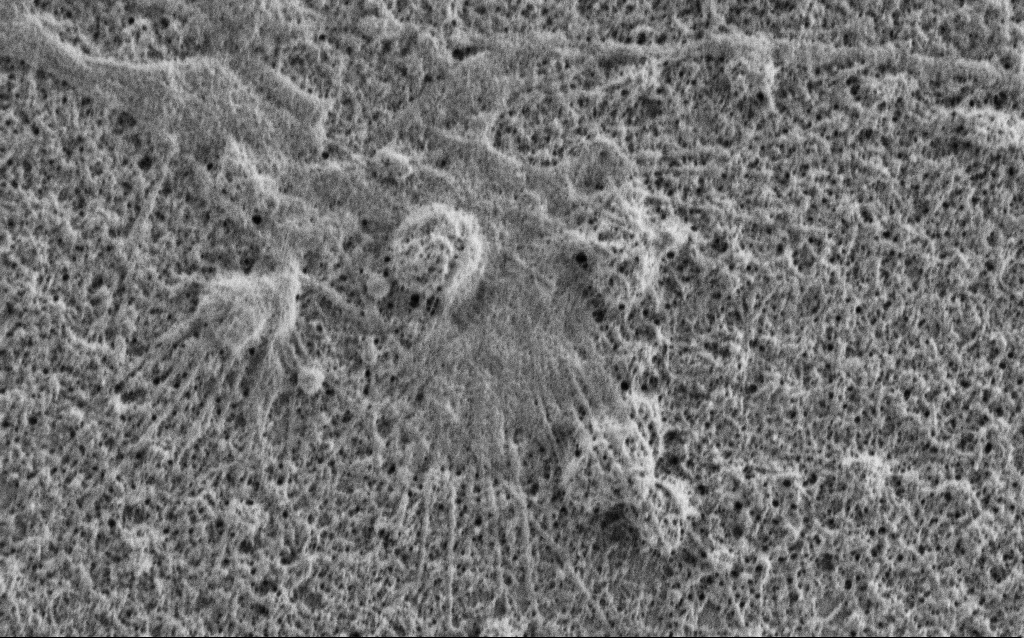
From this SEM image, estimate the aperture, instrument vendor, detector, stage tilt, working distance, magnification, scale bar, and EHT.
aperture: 30 µm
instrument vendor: Zeiss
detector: SE2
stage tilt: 0°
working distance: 5.3 mm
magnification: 20 K X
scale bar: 1000 nm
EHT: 1 kV